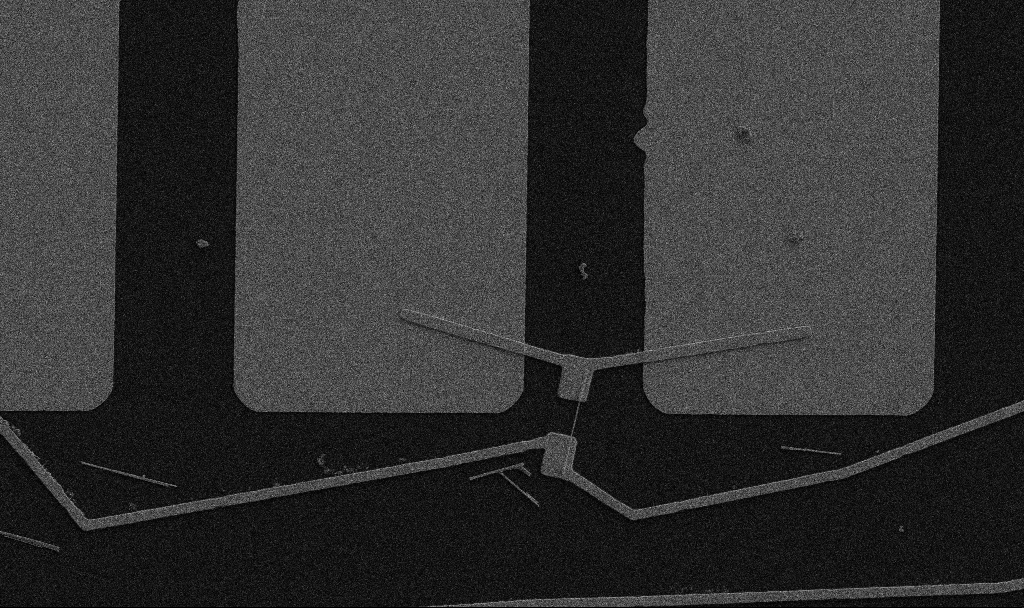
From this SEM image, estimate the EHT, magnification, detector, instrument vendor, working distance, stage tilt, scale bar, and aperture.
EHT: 5 kV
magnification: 5 K X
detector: SE2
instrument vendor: Zeiss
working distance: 10.7 mm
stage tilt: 0°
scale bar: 10000 nm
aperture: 30 µm